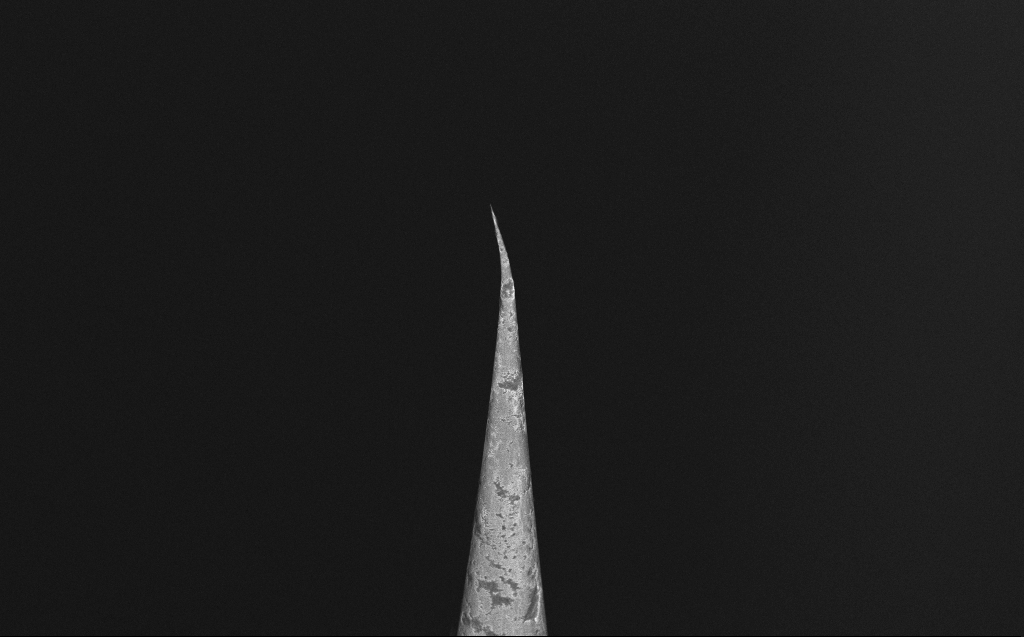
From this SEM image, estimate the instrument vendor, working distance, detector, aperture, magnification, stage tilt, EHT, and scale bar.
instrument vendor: Zeiss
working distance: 6 mm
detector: InLens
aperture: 30 µm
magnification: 3.81 K X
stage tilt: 40°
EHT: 10 kV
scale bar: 10000 nm